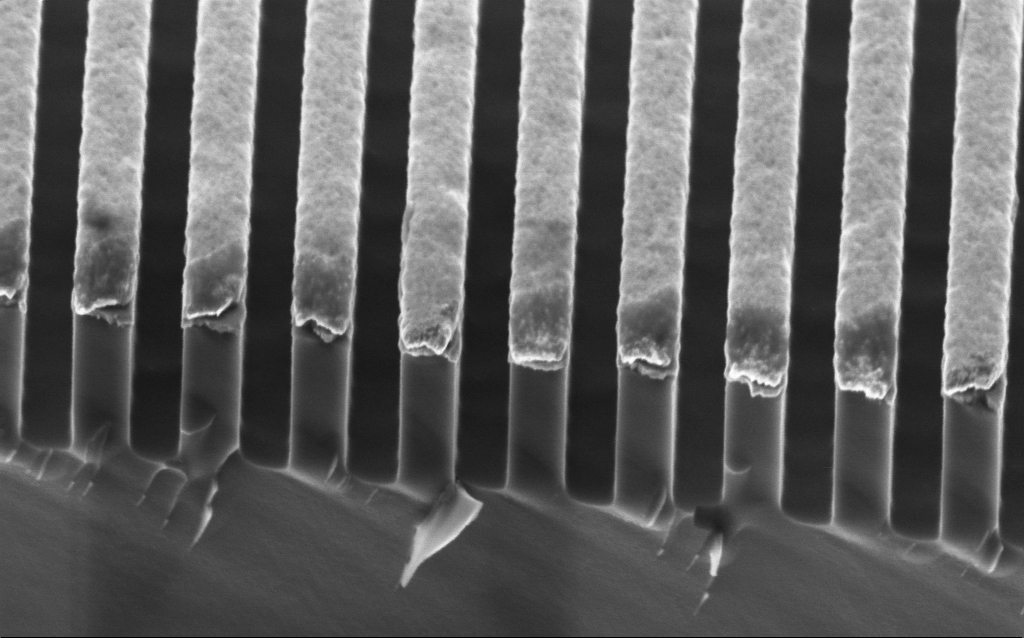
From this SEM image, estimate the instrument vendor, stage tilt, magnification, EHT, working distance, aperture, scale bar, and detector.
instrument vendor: Zeiss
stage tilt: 45°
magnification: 80.51 K X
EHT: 2 kV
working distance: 2.9 mm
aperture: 30 µm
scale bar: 200 nm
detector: InLens